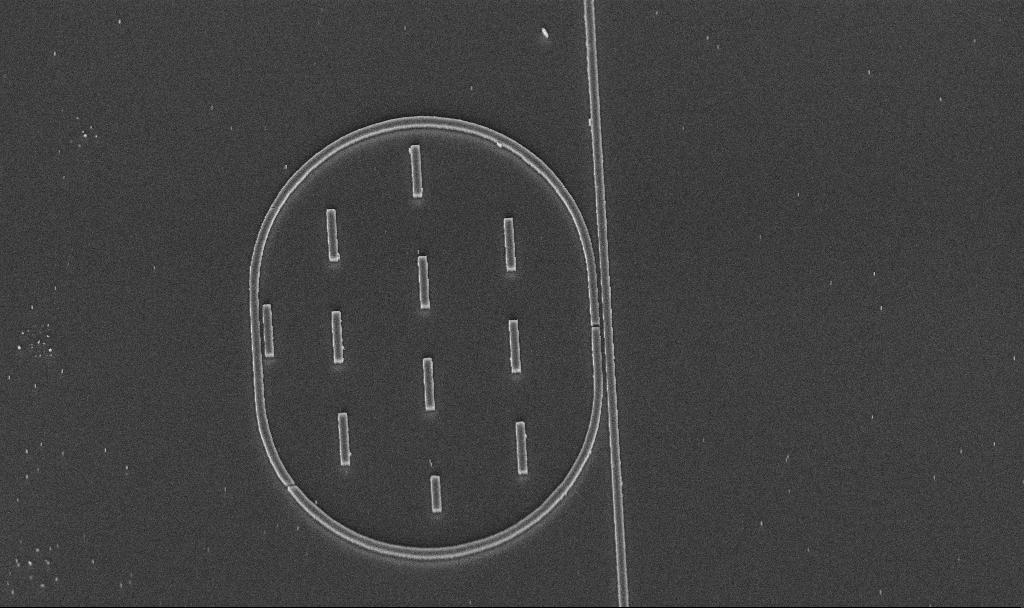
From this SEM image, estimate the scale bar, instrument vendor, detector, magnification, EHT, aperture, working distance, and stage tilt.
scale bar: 10000 nm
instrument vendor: Zeiss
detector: InLens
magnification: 6.38 K X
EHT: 5 kV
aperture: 30 µm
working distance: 9.8 mm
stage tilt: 45°